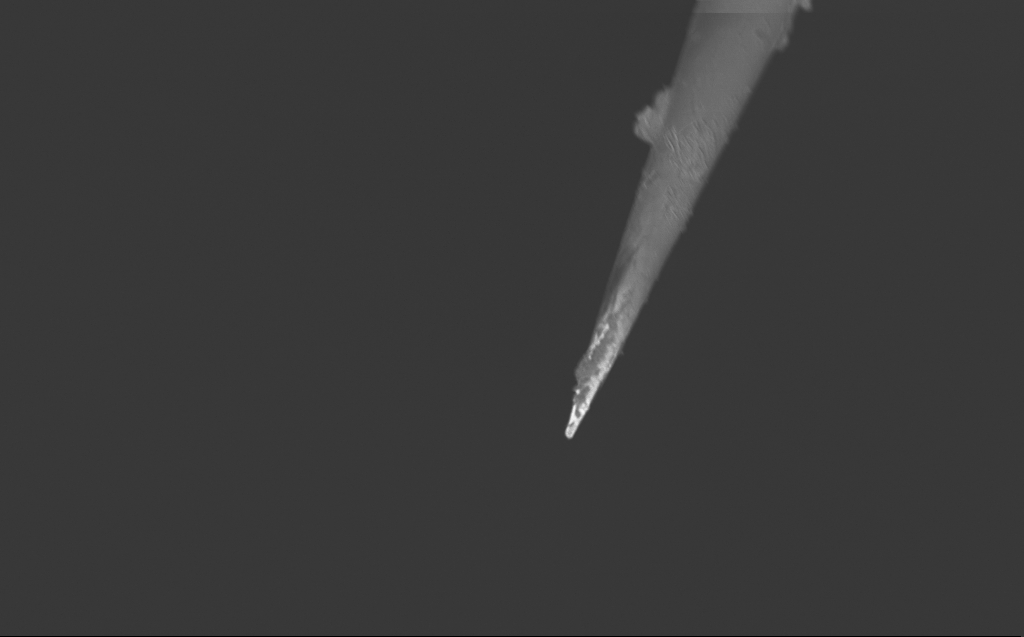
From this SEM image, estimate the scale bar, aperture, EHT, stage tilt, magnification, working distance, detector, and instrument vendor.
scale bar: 10000 nm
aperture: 30 µm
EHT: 2 kV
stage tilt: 45°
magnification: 5 K X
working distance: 3 mm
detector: InLens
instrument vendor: Zeiss